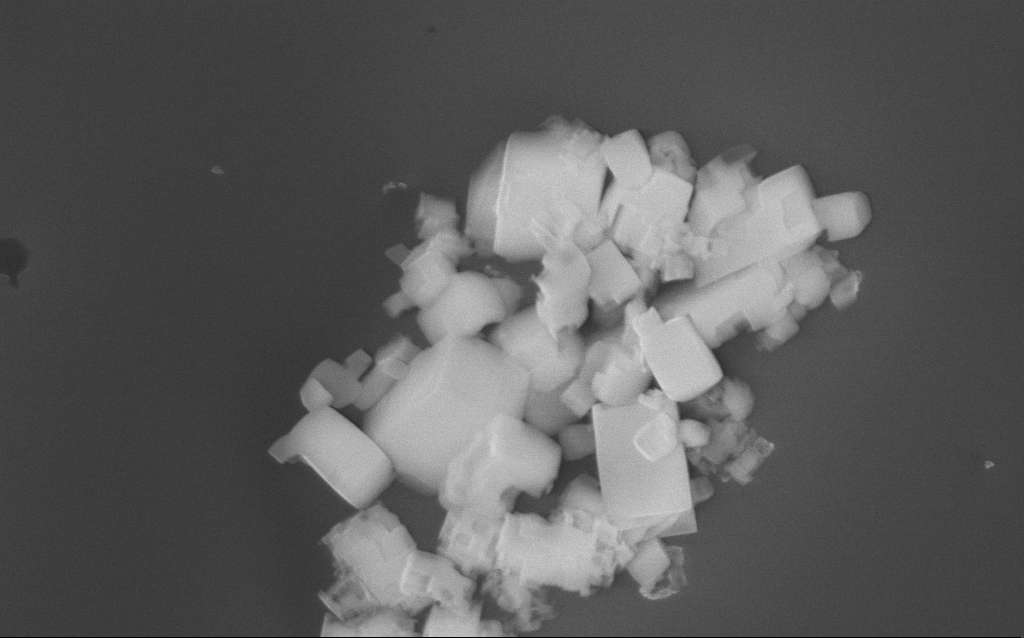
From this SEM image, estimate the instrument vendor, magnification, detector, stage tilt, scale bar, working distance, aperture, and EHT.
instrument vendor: Zeiss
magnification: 36.5 K X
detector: InLens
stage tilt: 0°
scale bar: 1000 nm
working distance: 2 mm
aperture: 30 µm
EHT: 10 kV